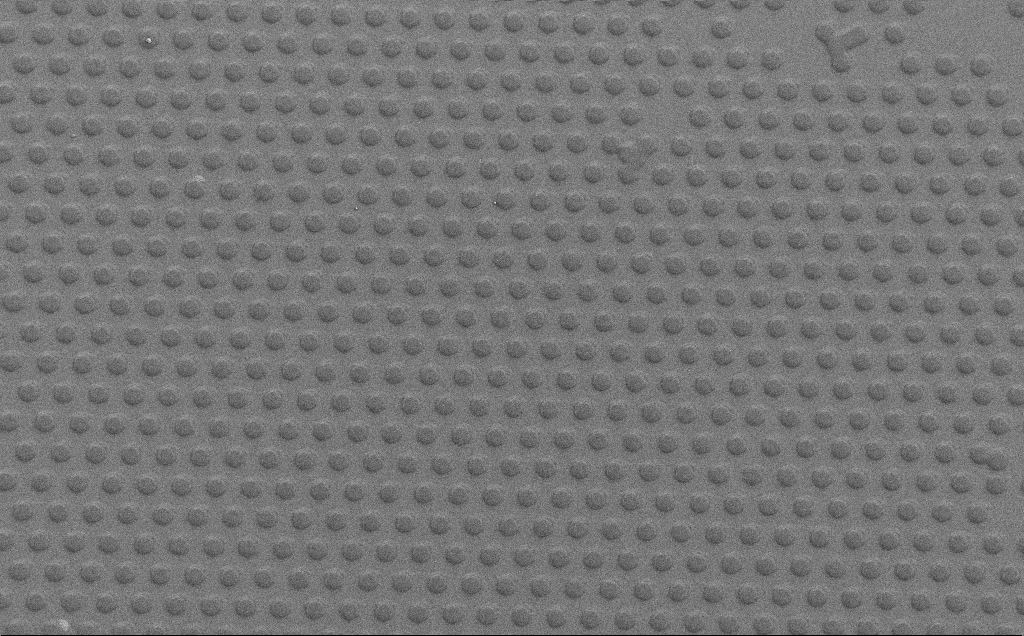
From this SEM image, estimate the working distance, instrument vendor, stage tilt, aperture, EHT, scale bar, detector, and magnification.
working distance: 5 mm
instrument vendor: Zeiss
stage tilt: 0°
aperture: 30 µm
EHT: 1.5 kV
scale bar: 100000 nm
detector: SE2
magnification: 0.635 K X